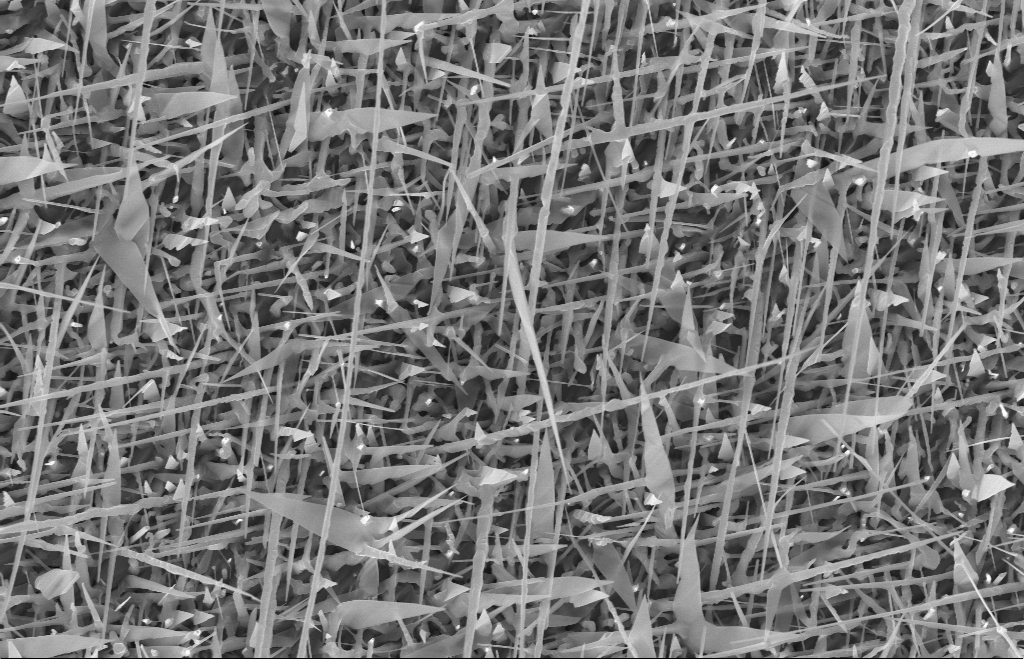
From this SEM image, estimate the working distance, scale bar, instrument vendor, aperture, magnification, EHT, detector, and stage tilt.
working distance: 10 mm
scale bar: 1000 nm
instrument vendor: Zeiss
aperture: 30 µm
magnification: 20 K X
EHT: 10 kV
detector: InLens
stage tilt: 0°